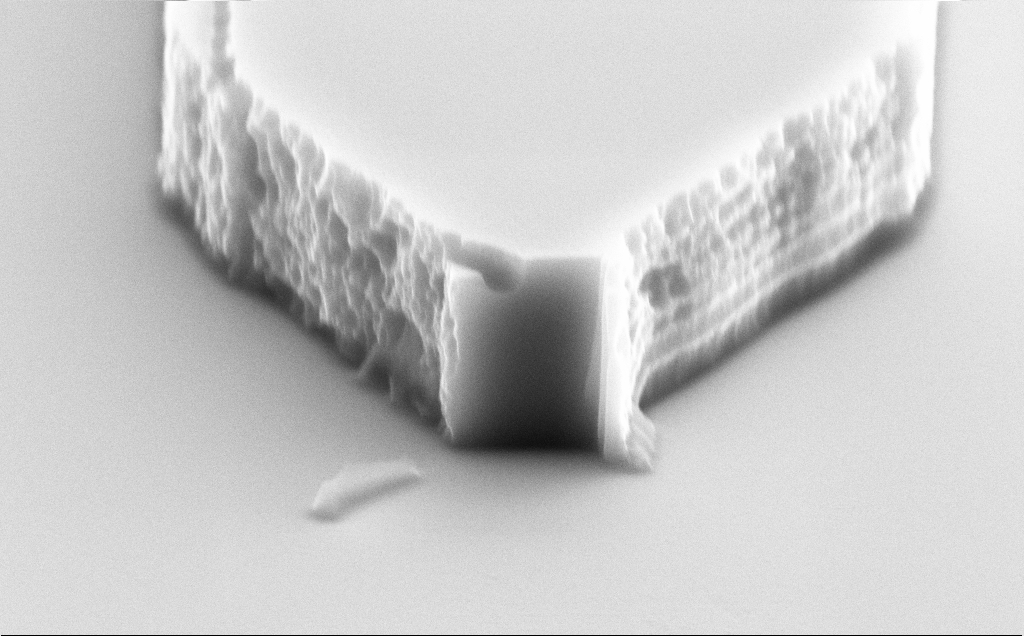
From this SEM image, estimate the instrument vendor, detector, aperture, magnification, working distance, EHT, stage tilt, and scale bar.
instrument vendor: Zeiss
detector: SE2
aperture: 30 µm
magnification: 29.98 K X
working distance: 9 mm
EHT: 10 kV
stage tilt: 70°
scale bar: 1000 nm